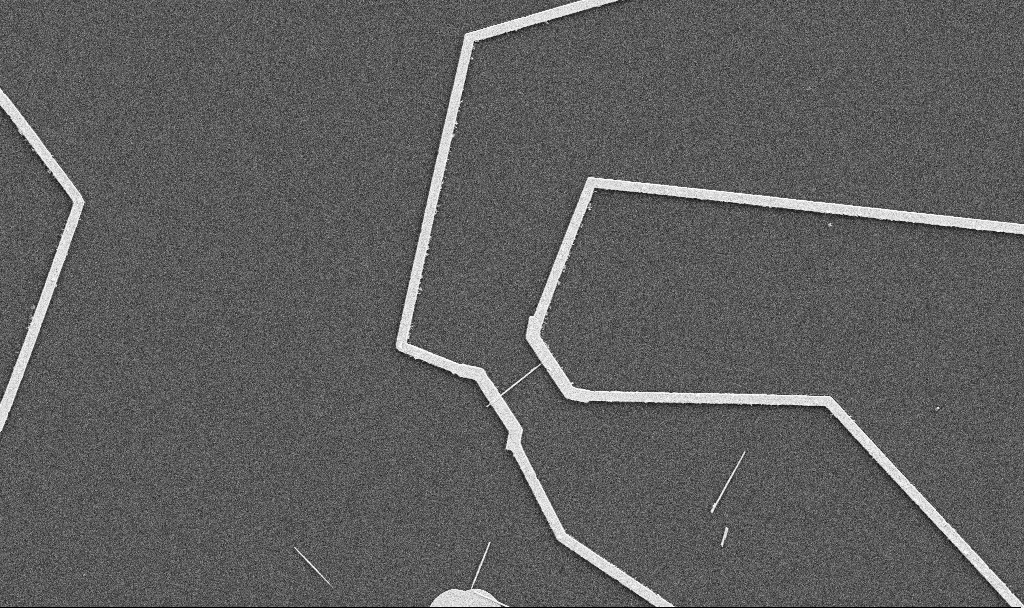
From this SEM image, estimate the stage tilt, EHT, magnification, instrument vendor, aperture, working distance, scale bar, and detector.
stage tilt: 0°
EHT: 5 kV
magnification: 5 K X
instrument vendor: Zeiss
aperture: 30 µm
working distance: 10.7 mm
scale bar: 10000 nm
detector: SE2